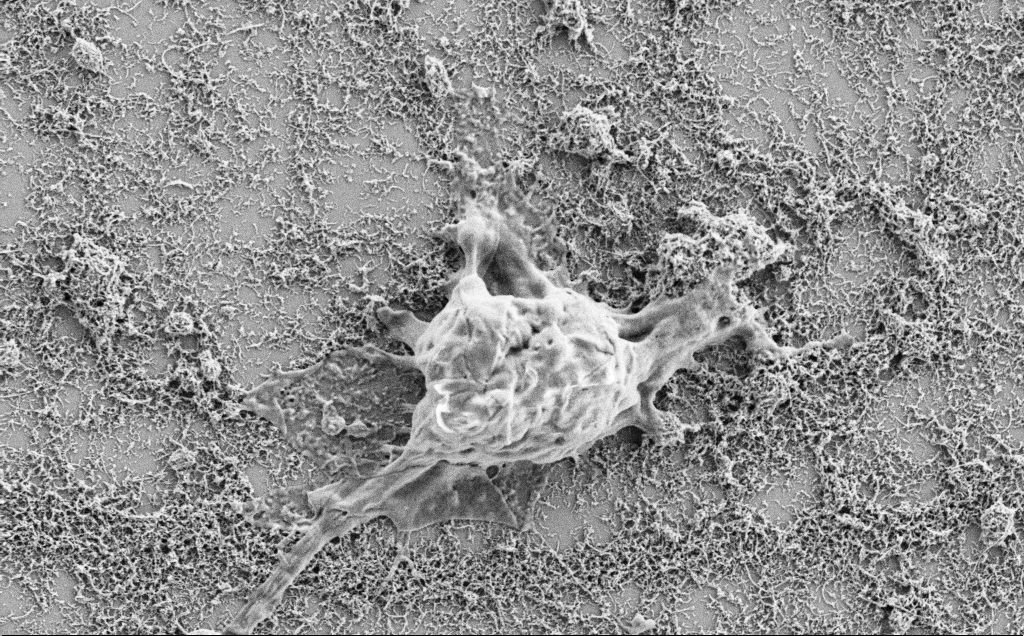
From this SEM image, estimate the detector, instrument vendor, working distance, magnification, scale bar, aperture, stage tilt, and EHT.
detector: SE2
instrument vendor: Zeiss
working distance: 7.1 mm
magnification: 10 K X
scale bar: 2000 nm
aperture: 30 µm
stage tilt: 0°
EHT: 2 kV